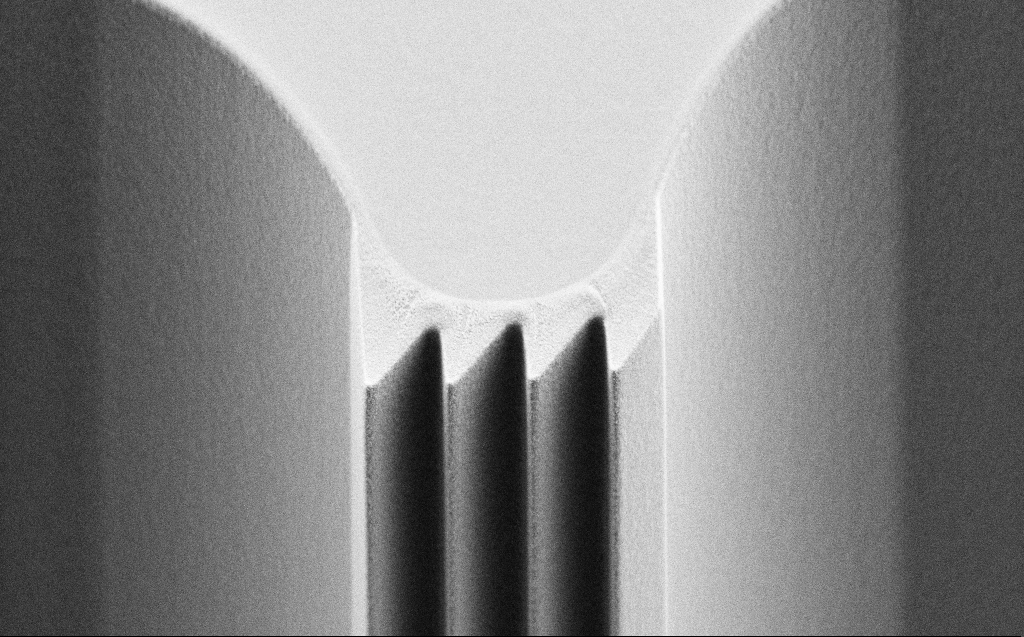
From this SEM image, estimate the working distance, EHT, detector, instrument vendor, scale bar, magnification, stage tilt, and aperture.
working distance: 6 mm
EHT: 5 kV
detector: SE2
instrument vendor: Zeiss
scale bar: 20000 nm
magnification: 2.67 K X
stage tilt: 45°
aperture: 30 µm